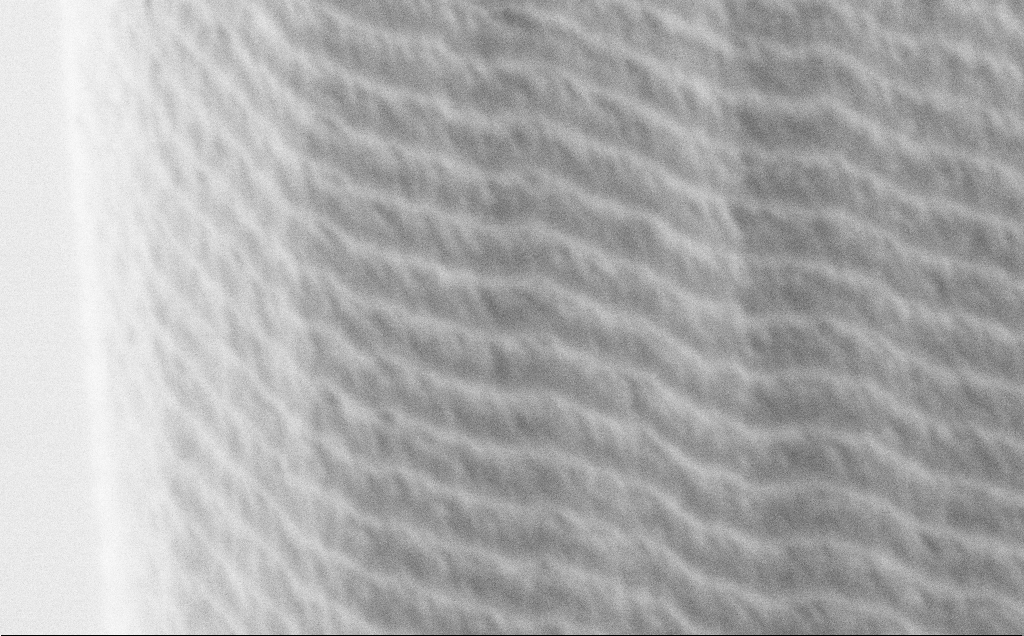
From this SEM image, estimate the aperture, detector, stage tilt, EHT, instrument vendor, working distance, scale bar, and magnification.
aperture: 30 µm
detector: SE2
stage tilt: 45°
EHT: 1.2 kV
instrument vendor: Zeiss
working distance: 8 mm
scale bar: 1000 nm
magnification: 58.09 K X